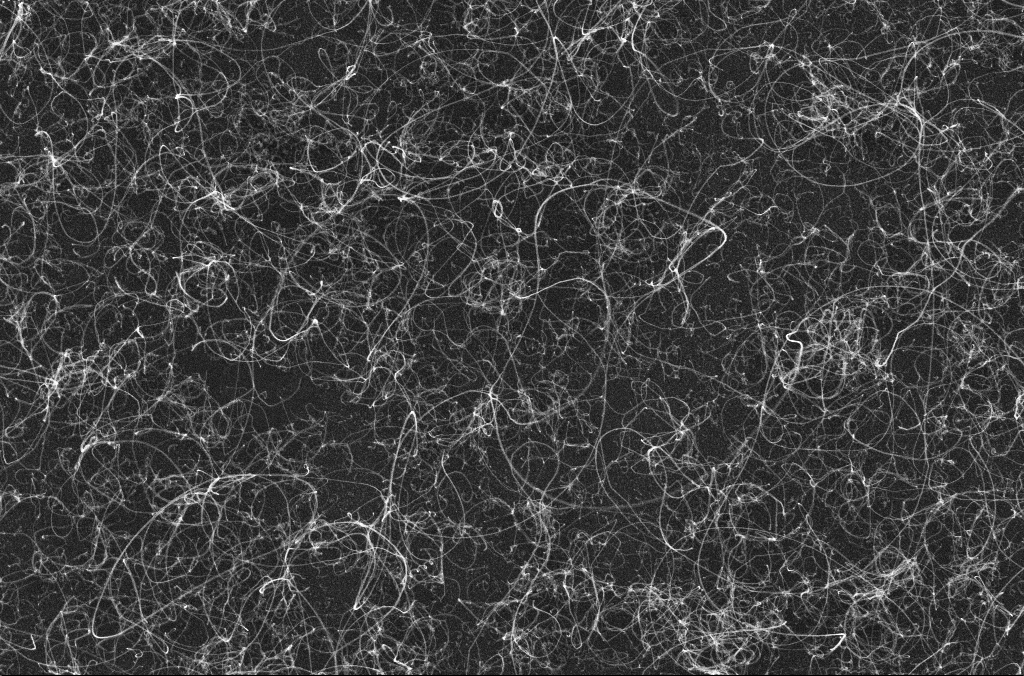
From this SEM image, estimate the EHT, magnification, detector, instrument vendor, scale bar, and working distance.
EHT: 10 kV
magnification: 50 K X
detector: InLens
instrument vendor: Zeiss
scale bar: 1000 nm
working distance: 3.2 mm